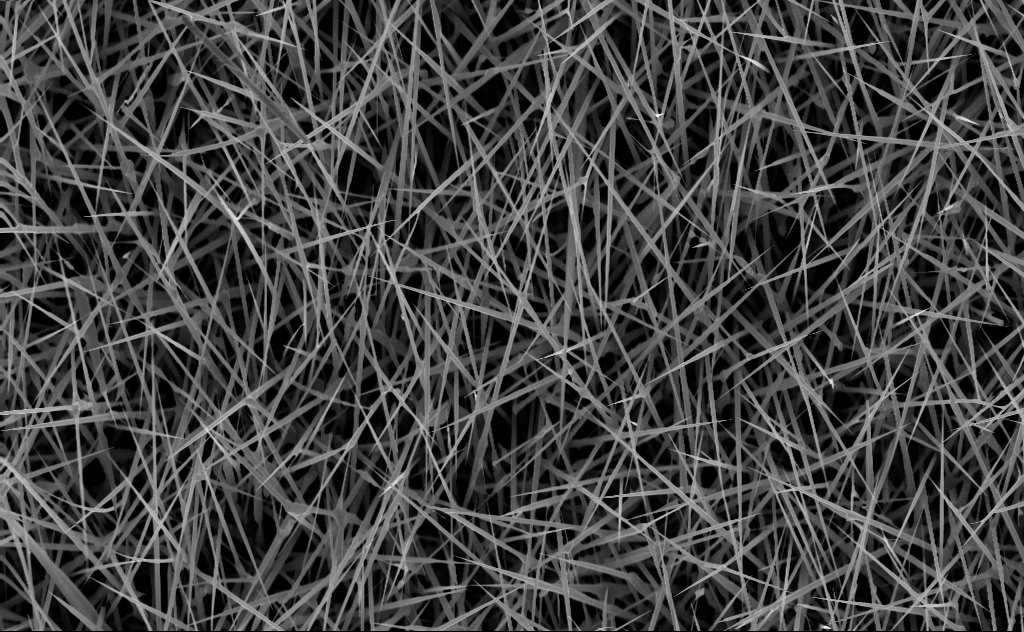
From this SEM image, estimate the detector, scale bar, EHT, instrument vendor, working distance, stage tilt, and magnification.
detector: InLens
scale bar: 2000 nm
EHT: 10 kV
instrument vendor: Zeiss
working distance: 5 mm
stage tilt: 0°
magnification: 10 K X